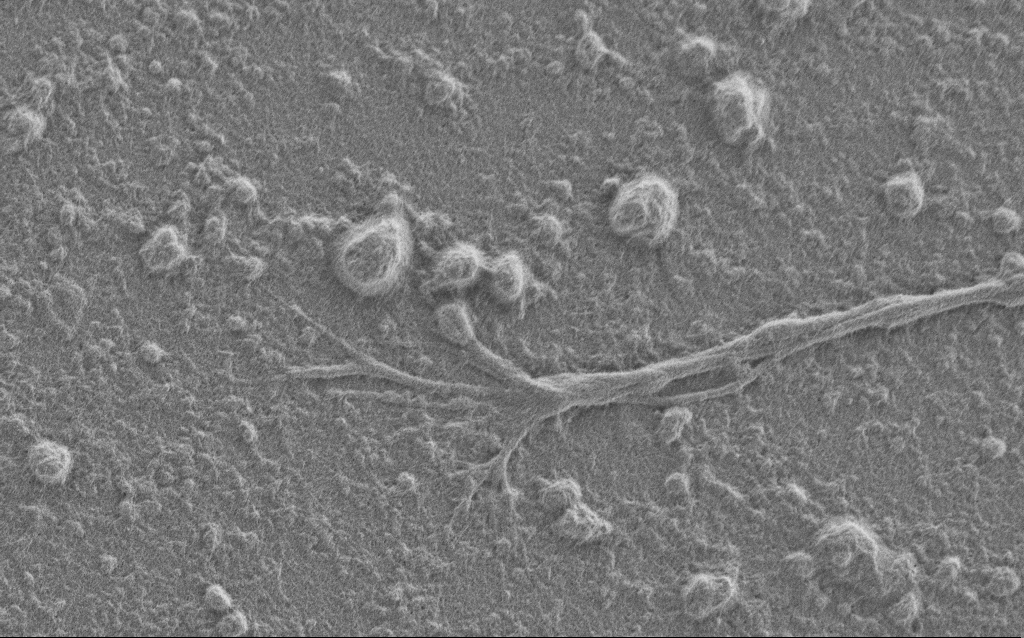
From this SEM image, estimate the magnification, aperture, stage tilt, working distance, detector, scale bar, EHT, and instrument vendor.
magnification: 7.5 K X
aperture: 30 µm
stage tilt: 0°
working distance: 6 mm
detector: SE2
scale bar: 2000 nm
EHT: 1 kV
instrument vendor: Zeiss